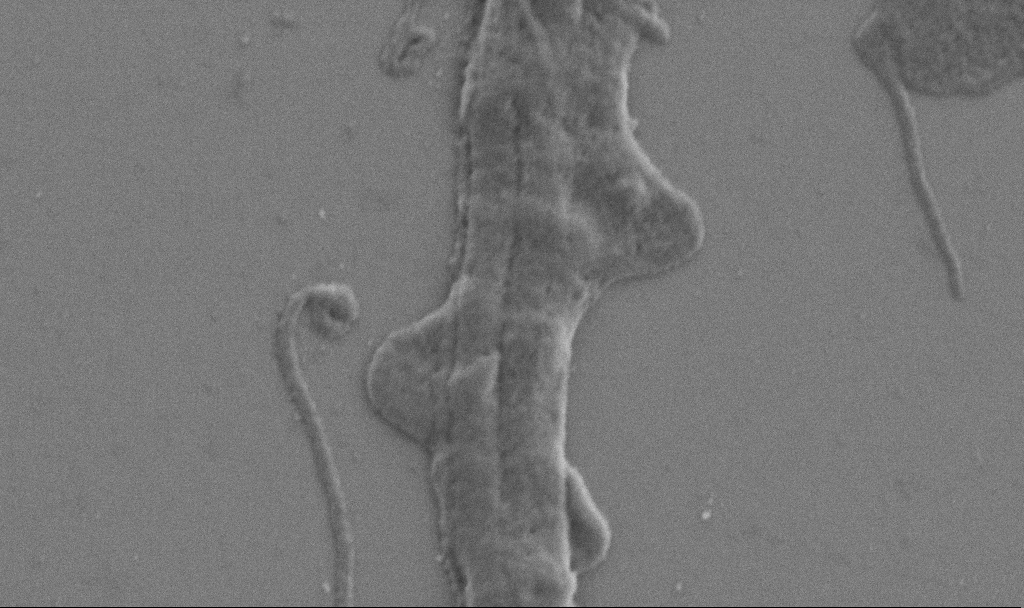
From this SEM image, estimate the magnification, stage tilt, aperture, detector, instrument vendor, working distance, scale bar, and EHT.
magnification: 40 K X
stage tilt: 0°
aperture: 30 µm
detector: SE2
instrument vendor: Zeiss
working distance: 6.9 mm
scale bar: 1000 nm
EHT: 1 kV